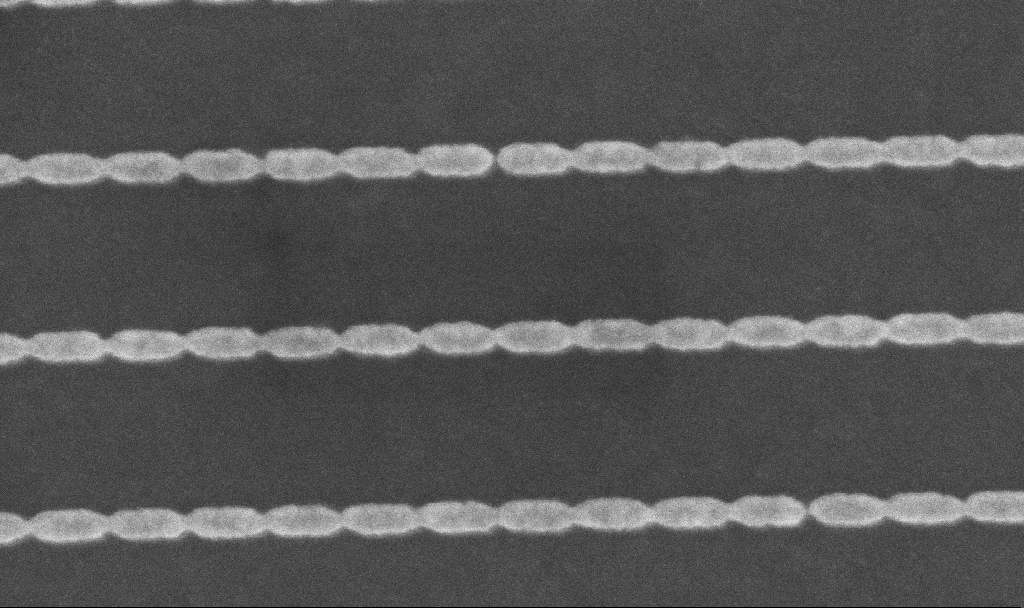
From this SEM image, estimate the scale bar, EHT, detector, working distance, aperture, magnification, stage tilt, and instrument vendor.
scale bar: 200 nm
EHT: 5 kV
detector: InLens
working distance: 6 mm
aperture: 30 µm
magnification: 219.21 K X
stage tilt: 0°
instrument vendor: Zeiss